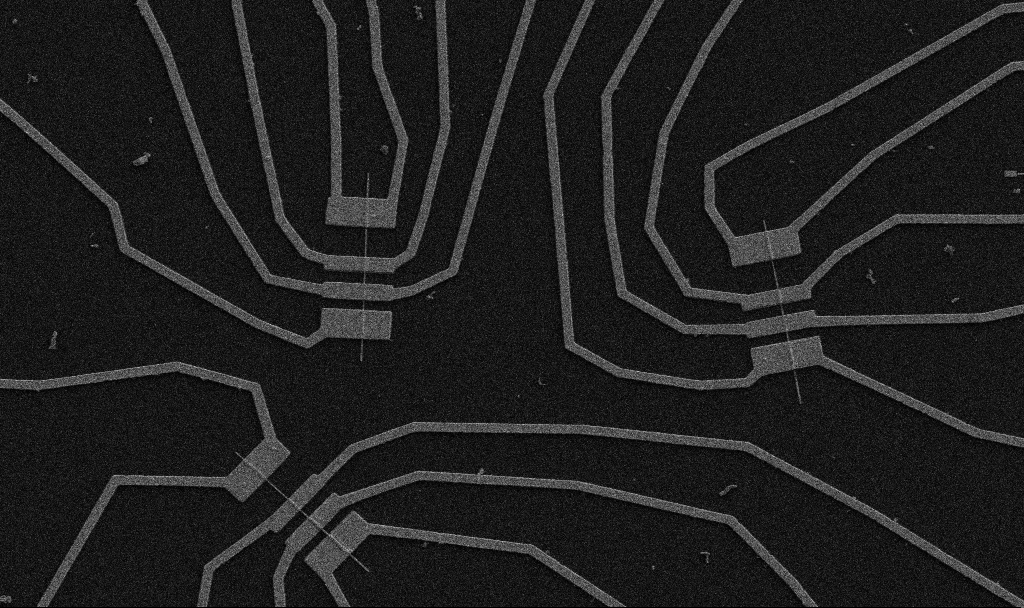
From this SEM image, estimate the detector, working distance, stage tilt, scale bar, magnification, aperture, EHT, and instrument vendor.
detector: SE2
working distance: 10.7 mm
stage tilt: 0°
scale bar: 10000 nm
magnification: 5 K X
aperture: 30 µm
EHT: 5 kV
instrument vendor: Zeiss